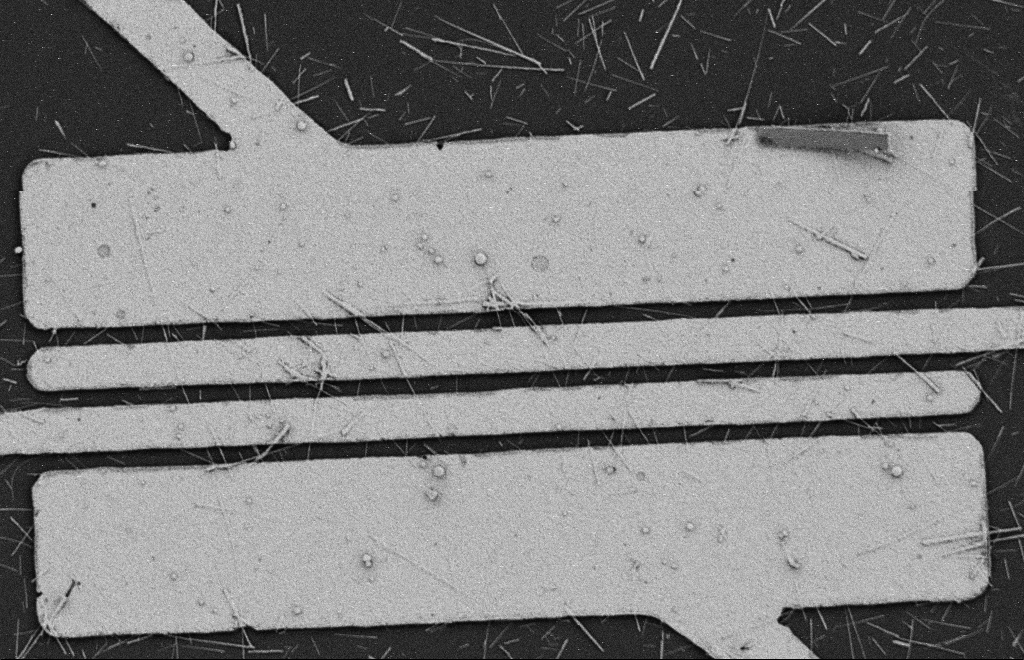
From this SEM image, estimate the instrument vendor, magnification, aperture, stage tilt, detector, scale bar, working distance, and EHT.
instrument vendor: Zeiss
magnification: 5.68 K X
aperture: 20 µm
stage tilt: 0°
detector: SE2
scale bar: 2000 nm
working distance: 9 mm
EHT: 2 kV